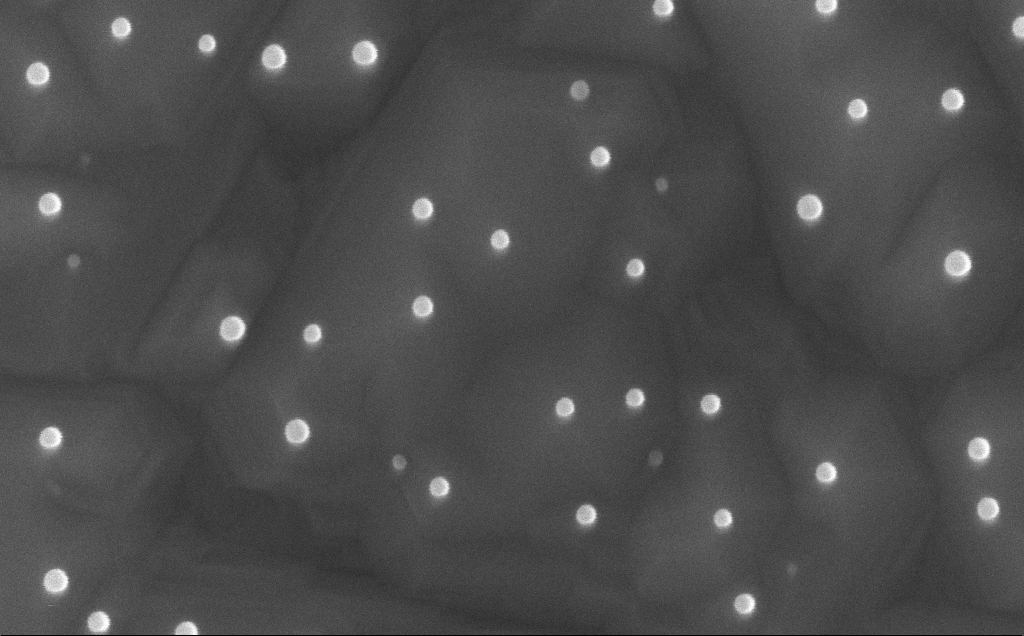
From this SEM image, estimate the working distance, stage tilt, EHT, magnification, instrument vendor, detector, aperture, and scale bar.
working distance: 6 mm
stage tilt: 0°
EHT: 10 kV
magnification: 200 K X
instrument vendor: Zeiss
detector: InLens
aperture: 30 µm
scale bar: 100 nm